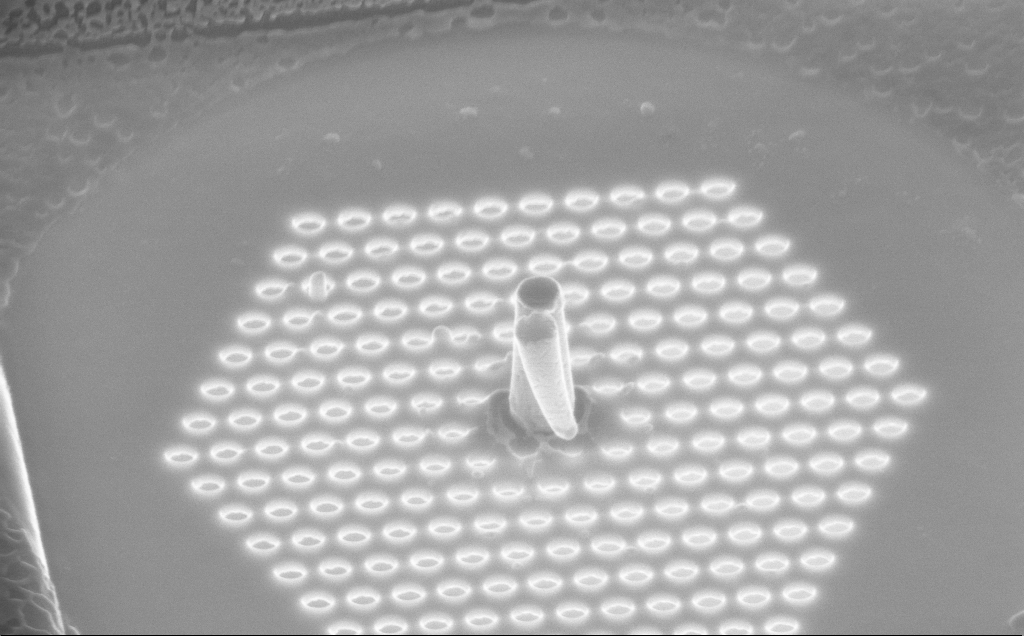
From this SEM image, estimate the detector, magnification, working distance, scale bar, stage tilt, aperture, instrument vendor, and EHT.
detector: InLens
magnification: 67.83 K X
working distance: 8 mm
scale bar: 1000 nm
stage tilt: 45°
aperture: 30 µm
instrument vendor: Zeiss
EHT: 10 kV